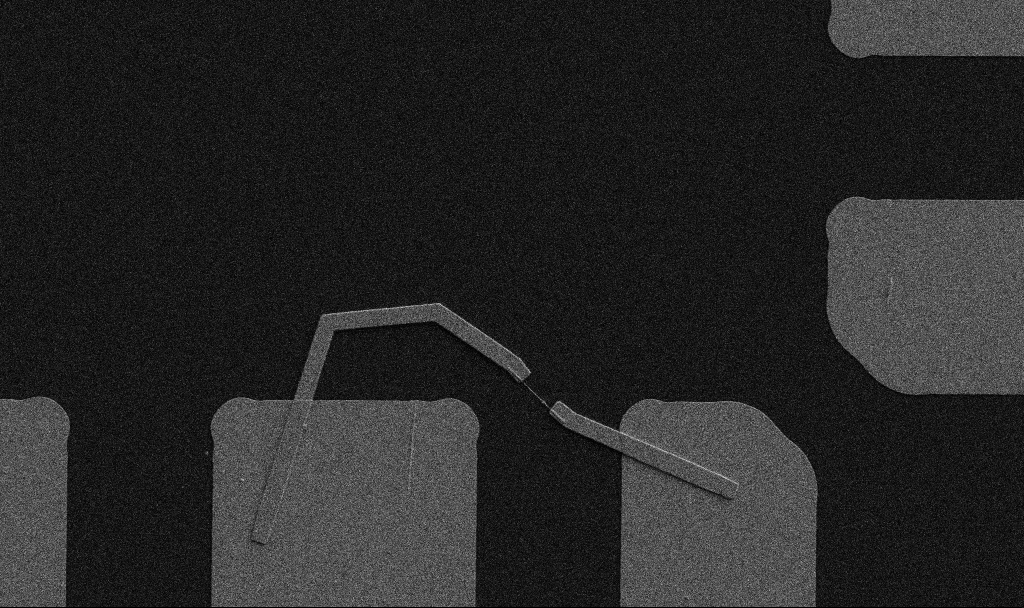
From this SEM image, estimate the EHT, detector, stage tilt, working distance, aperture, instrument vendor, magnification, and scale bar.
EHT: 5 kV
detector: SE2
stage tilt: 0°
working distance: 10.7 mm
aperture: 30 µm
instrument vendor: Zeiss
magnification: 5 K X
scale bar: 10000 nm